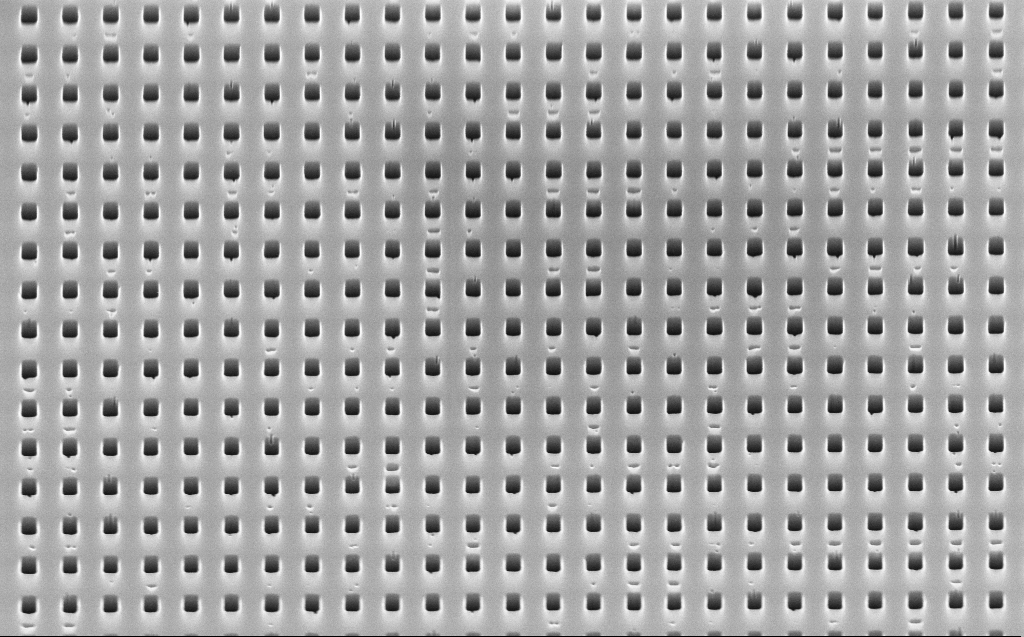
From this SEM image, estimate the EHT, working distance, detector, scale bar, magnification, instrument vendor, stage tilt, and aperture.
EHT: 10 kV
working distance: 4 mm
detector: InLens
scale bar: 2000 nm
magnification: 30 K X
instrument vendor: Zeiss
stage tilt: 45°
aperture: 30 µm